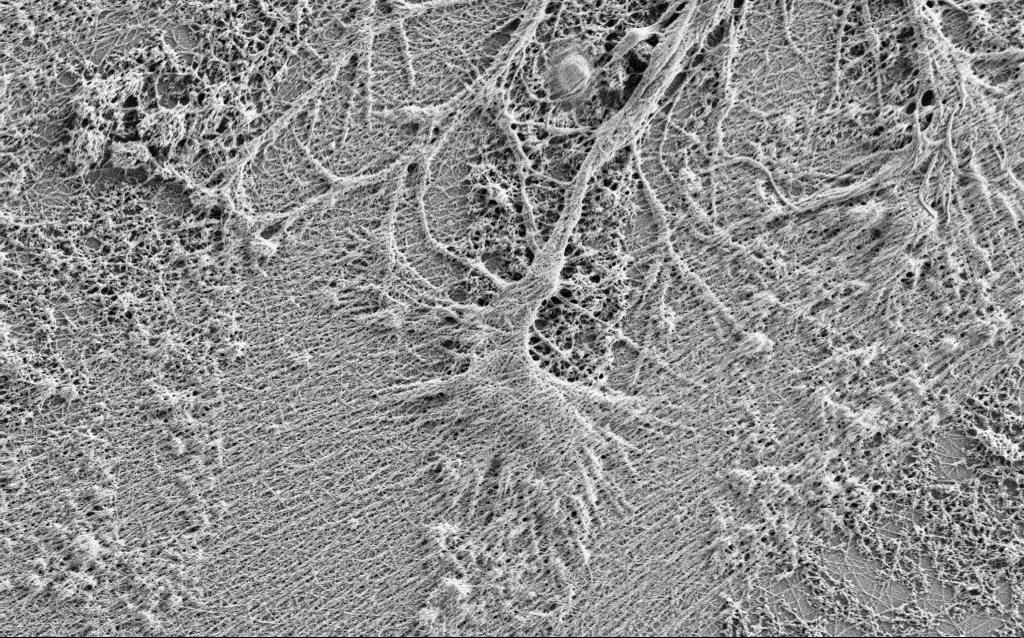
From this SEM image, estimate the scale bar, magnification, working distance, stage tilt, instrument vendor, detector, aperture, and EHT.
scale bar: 2000 nm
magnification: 10 K X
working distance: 4 mm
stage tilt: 0°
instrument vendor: Zeiss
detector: SE2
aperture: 30 µm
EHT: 1 kV